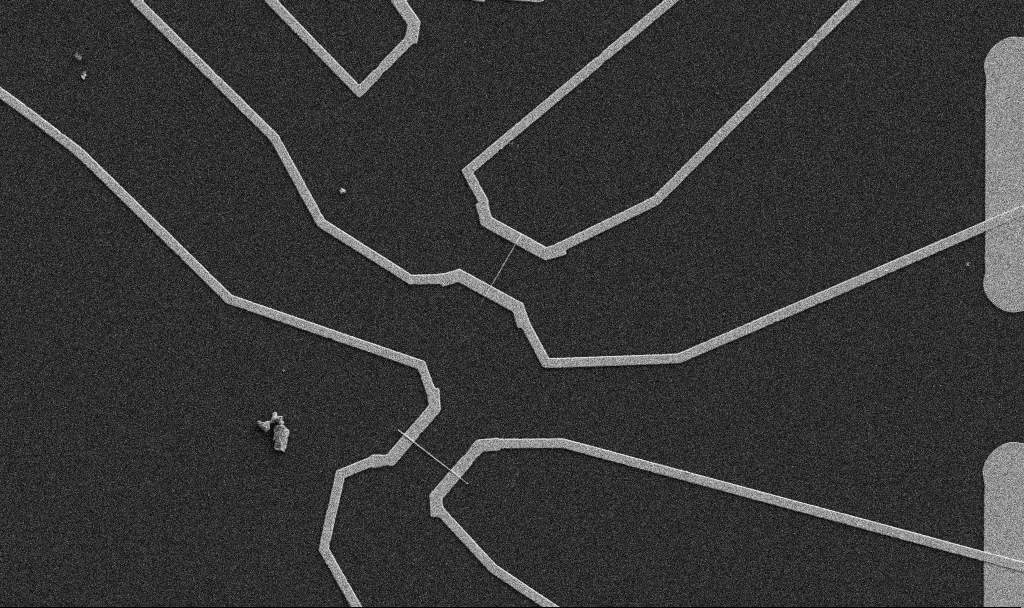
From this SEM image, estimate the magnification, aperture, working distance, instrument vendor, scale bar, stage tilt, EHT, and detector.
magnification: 5 K X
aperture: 30 µm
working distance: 10.7 mm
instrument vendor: Zeiss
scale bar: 10000 nm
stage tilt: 0°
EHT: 5 kV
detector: SE2